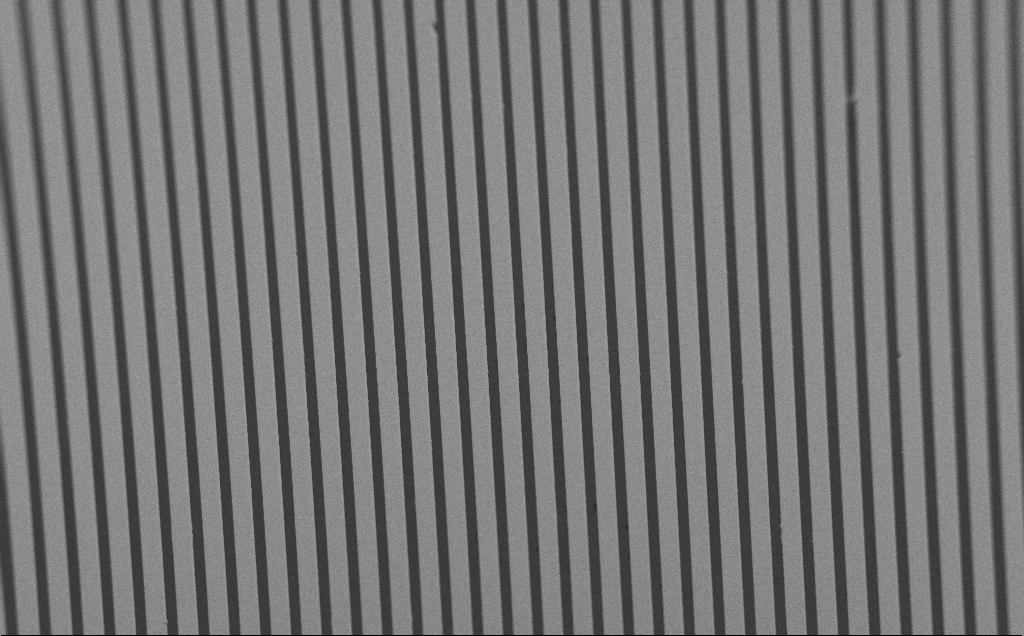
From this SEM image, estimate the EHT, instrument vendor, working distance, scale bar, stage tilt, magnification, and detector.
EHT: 1.2 kV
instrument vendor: Zeiss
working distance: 6 mm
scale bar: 100000 nm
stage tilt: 45°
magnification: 0.345 K X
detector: SE2